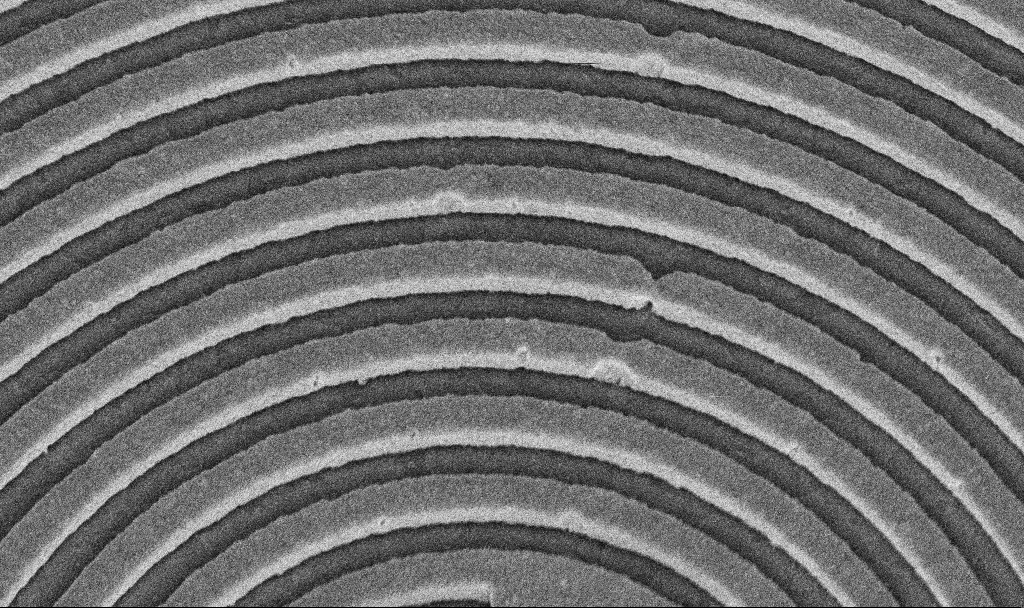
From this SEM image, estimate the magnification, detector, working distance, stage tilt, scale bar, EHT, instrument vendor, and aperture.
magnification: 46.54 K X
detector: InLens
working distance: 8.7 mm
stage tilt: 45°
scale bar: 1000 nm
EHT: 5 kV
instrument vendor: Zeiss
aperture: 30 µm